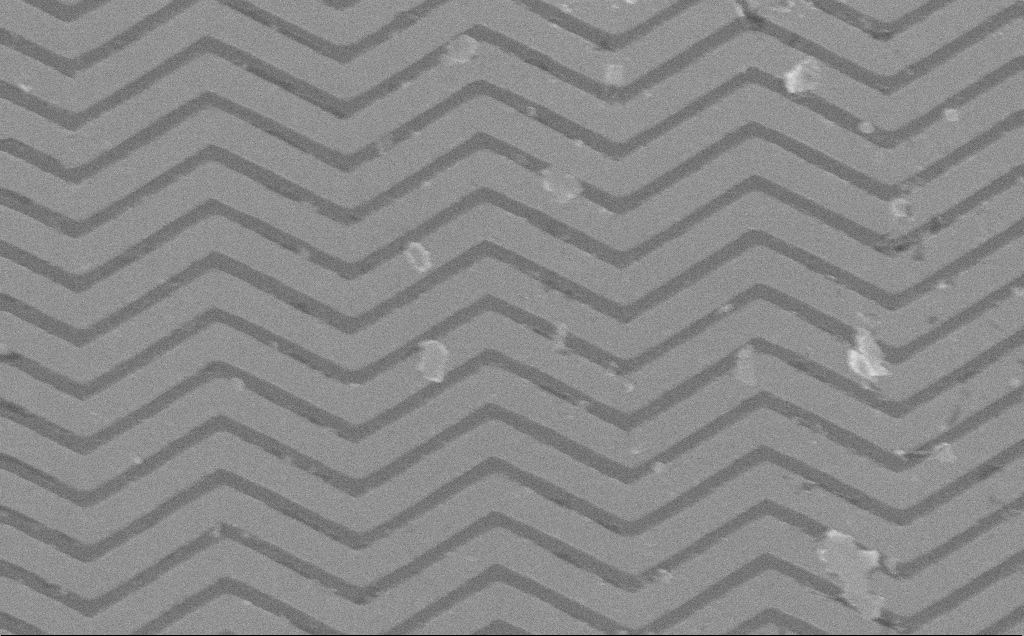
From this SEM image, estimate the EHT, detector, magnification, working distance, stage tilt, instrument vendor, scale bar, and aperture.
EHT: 10 kV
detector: InLens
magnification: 49.86 K X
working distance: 7 mm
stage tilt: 0°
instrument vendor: Zeiss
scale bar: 1000 nm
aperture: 30 µm